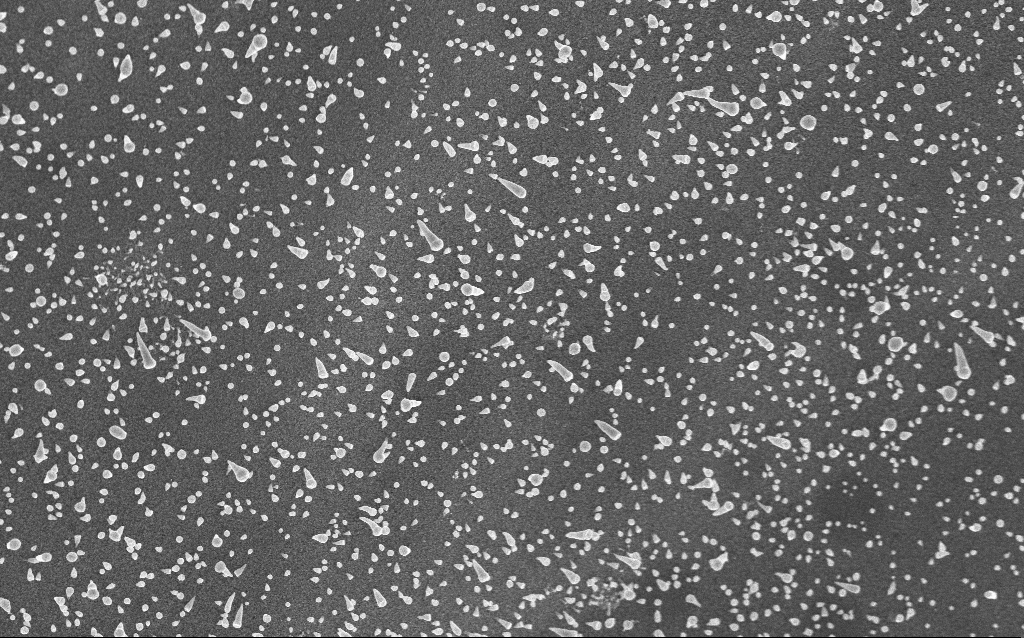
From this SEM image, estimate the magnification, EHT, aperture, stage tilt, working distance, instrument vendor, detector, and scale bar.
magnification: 20 K X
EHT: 5 kV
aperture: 30 µm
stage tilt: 0°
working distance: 5 mm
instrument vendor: Zeiss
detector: InLens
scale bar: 2000 nm